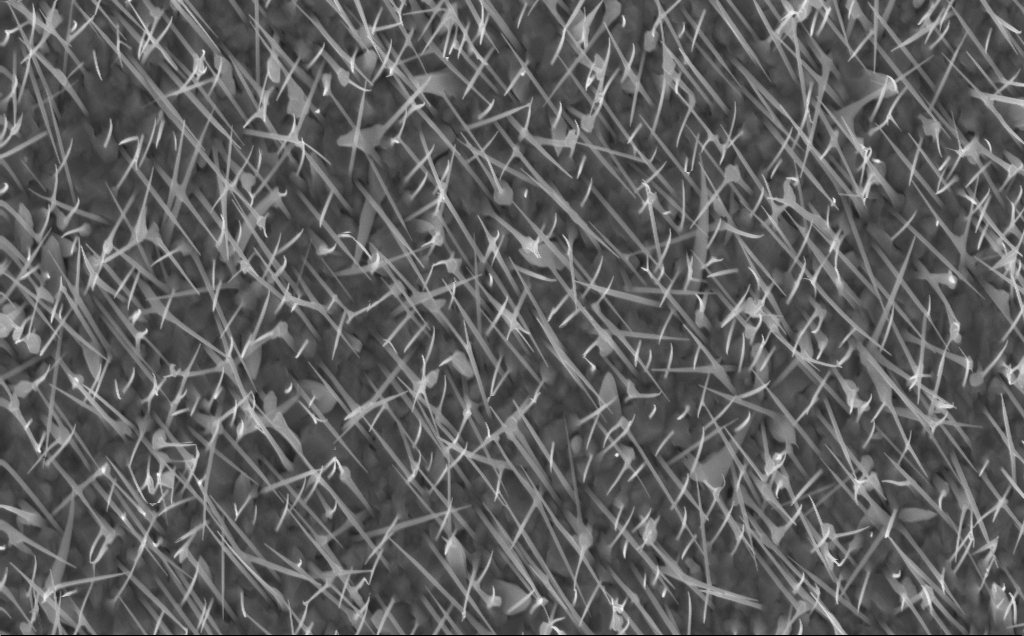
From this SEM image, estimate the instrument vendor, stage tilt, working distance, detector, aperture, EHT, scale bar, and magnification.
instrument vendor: Zeiss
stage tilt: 0°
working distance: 5 mm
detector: InLens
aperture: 30 µm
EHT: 10 kV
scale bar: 2000 nm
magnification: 20 K X